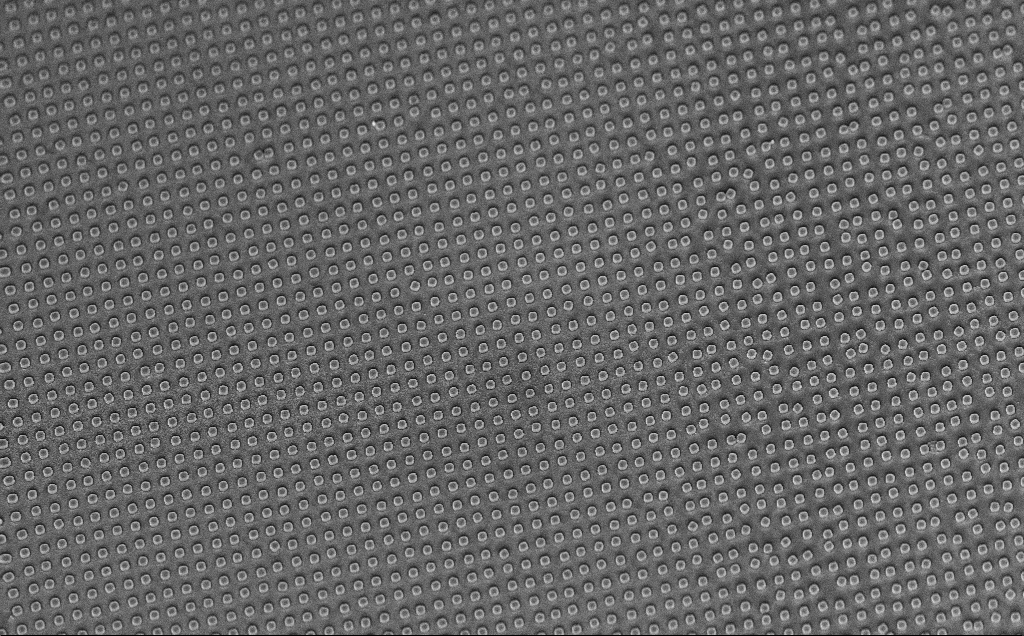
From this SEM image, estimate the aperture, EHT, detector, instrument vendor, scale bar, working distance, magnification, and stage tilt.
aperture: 30 µm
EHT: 5 kV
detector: InLens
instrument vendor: Zeiss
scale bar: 10000 nm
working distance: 7 mm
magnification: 4.59 K X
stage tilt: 45°